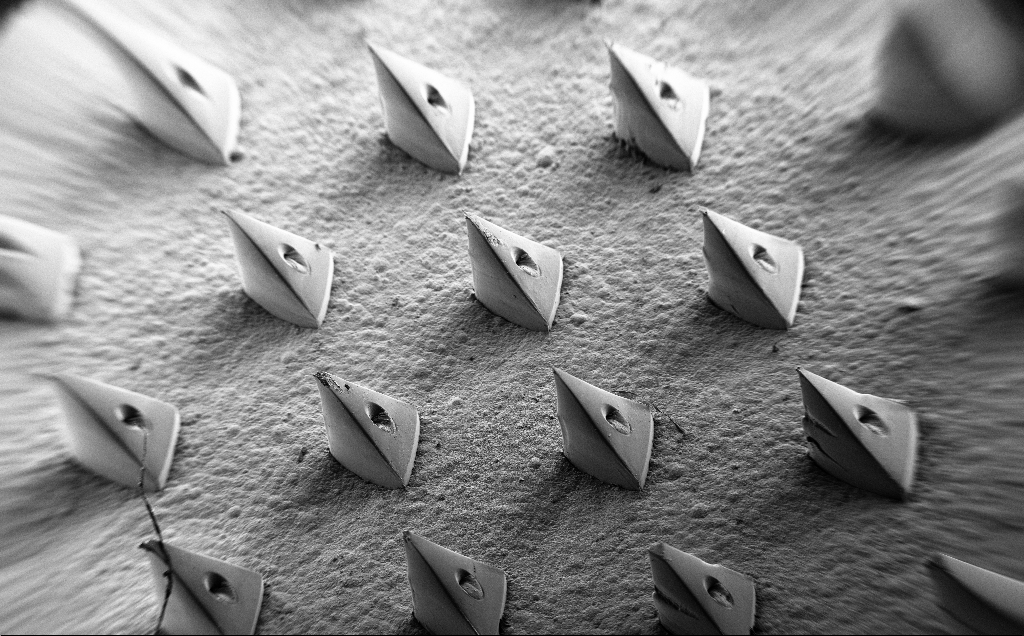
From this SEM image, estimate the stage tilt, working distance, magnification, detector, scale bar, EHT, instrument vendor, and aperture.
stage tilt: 35°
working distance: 12 mm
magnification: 0.058 K X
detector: SE2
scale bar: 1e+06 nm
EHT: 5 kV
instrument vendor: Zeiss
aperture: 30 µm